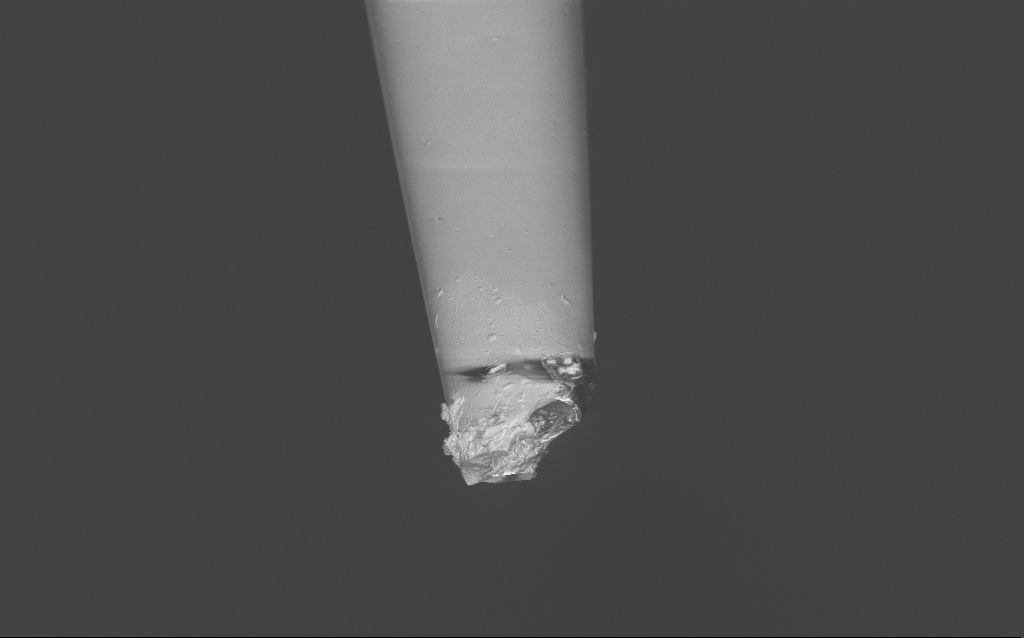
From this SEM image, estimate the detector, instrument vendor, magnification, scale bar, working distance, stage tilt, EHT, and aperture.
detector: InLens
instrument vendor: Zeiss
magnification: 5 K X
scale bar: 10000 nm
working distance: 6 mm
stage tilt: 45°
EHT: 2 kV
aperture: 30 µm